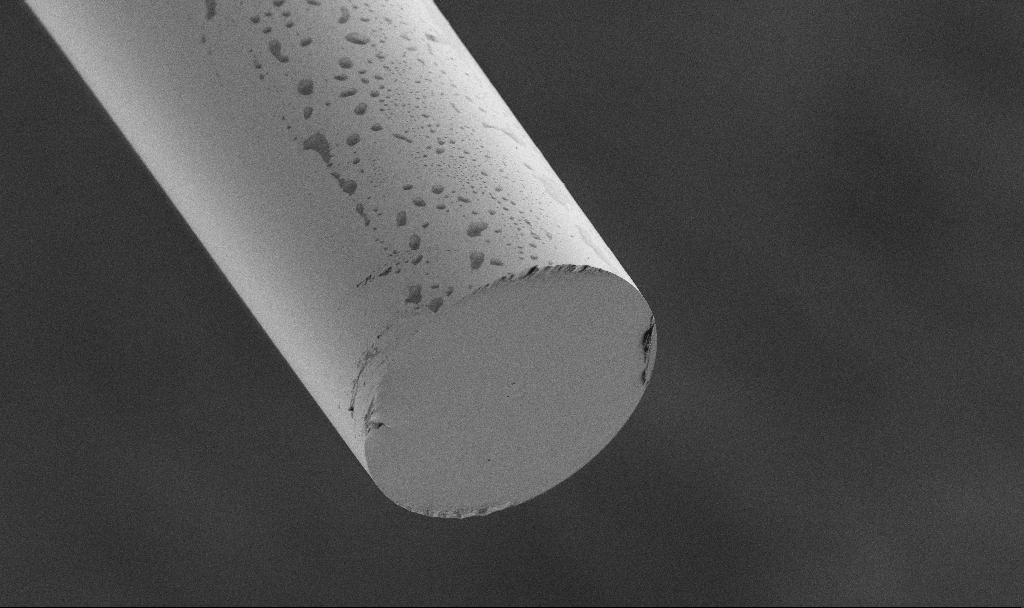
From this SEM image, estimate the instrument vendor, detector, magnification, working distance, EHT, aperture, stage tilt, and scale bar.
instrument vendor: Zeiss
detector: SE2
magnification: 0.947 K X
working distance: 3.3 mm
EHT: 1.5 kV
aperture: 30 µm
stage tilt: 45°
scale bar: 20000 nm